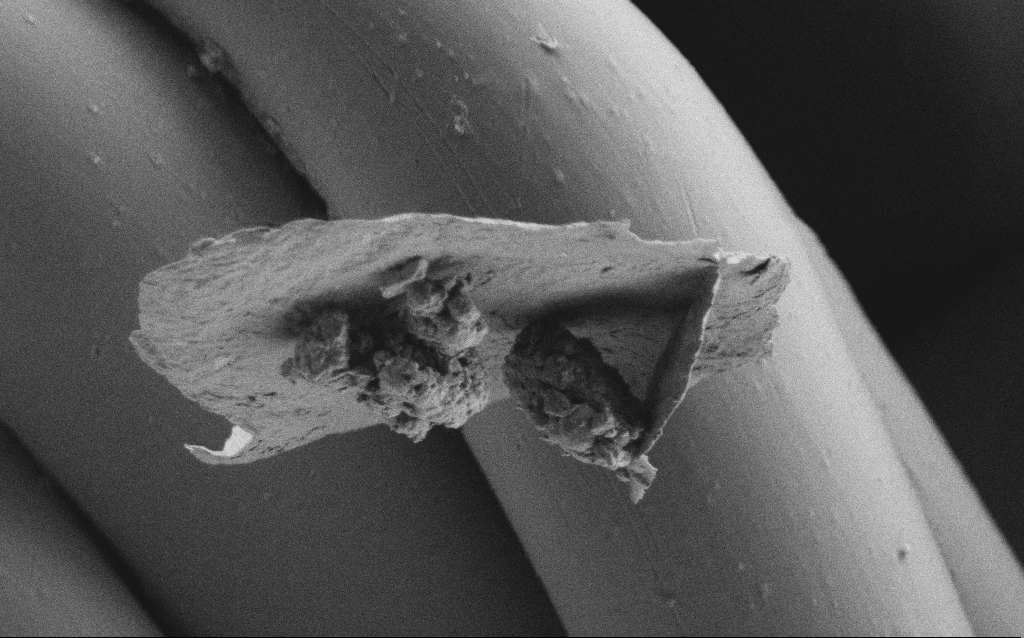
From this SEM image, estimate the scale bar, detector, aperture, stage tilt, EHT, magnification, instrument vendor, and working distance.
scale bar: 10000 nm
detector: SE2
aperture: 30 µm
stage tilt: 0°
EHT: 1 kV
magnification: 5.69 K X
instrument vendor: Zeiss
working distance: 4 mm